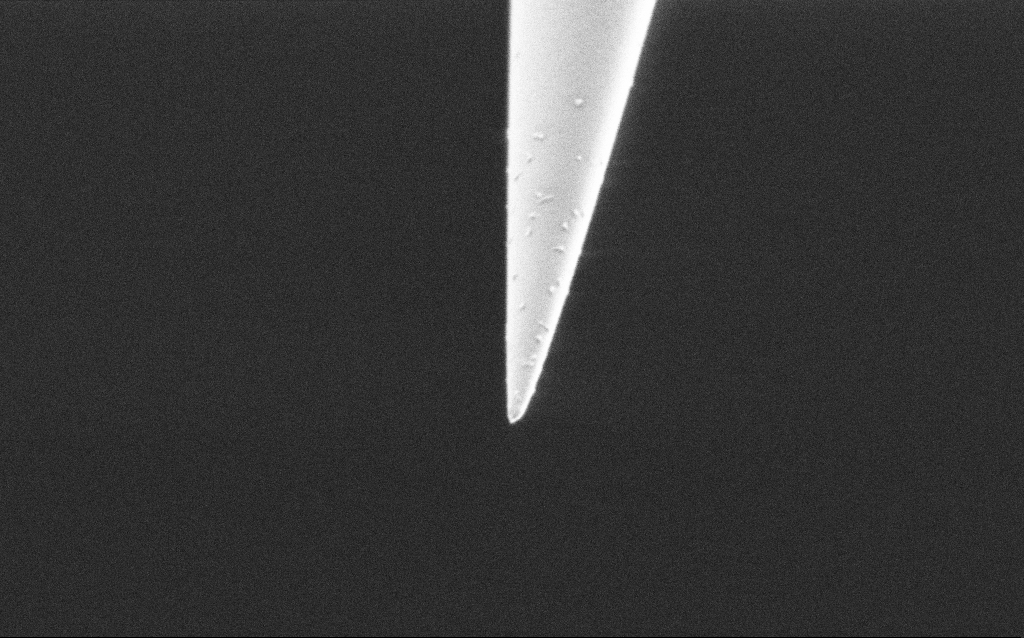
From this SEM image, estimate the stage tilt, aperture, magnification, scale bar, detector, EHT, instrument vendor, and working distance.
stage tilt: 45°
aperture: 30 µm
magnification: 50 K X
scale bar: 1000 nm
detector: SE2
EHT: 1 kV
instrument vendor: Zeiss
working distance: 6.4 mm